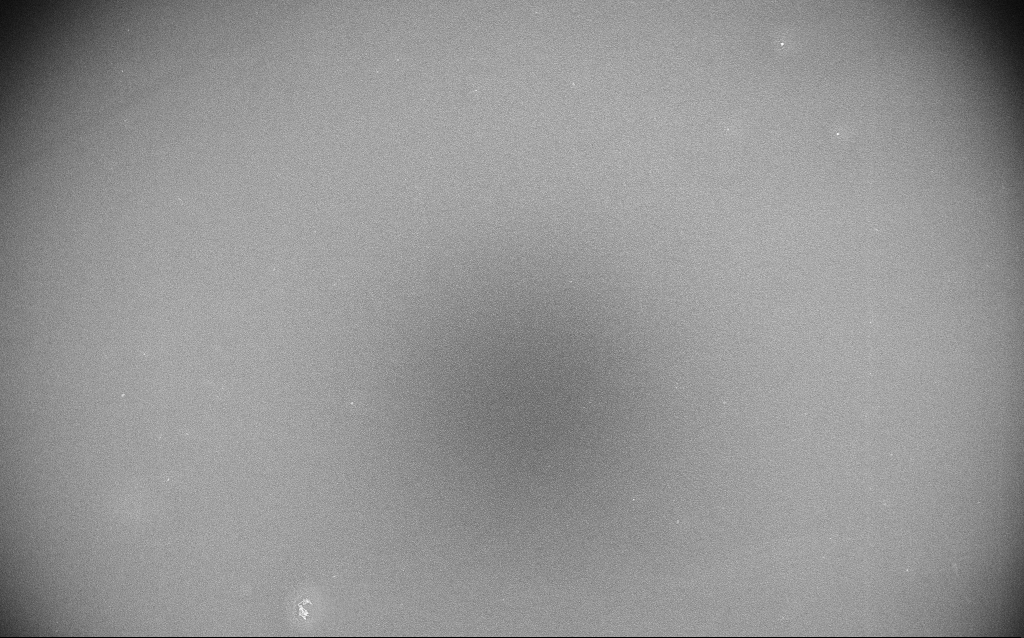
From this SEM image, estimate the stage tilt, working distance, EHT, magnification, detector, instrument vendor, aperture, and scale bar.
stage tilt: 0°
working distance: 1.5 mm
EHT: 20 kV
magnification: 0.2 K X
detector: InLens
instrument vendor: Zeiss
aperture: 30 µm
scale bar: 100000 nm